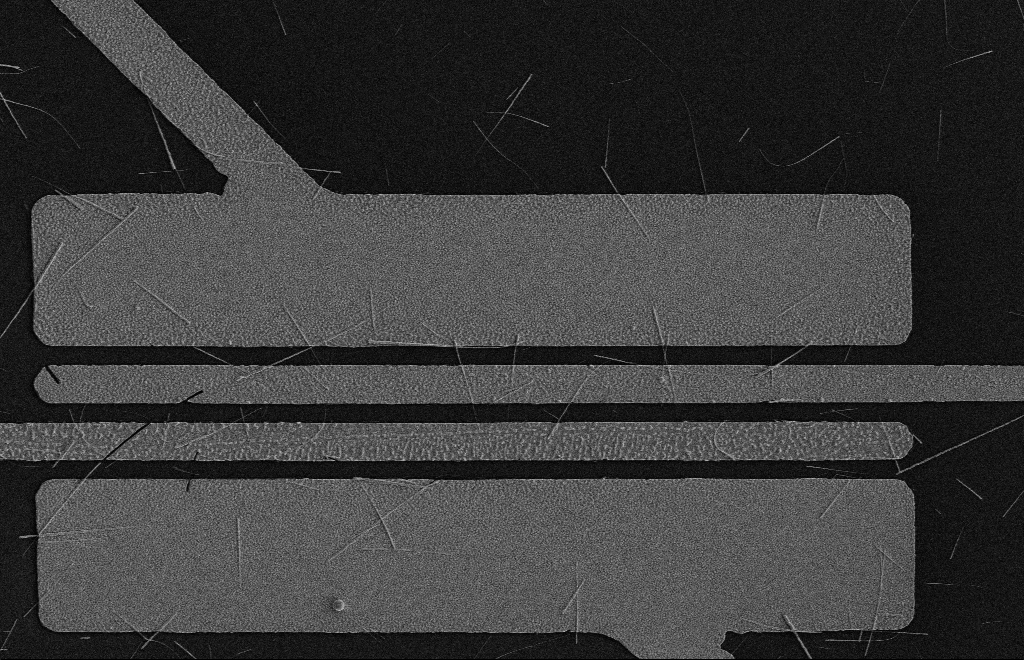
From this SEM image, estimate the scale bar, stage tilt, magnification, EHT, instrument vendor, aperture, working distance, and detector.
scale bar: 2000 nm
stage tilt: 0°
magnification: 5.31 K X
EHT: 5 kV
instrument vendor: Zeiss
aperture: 10 µm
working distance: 16 mm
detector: SE2